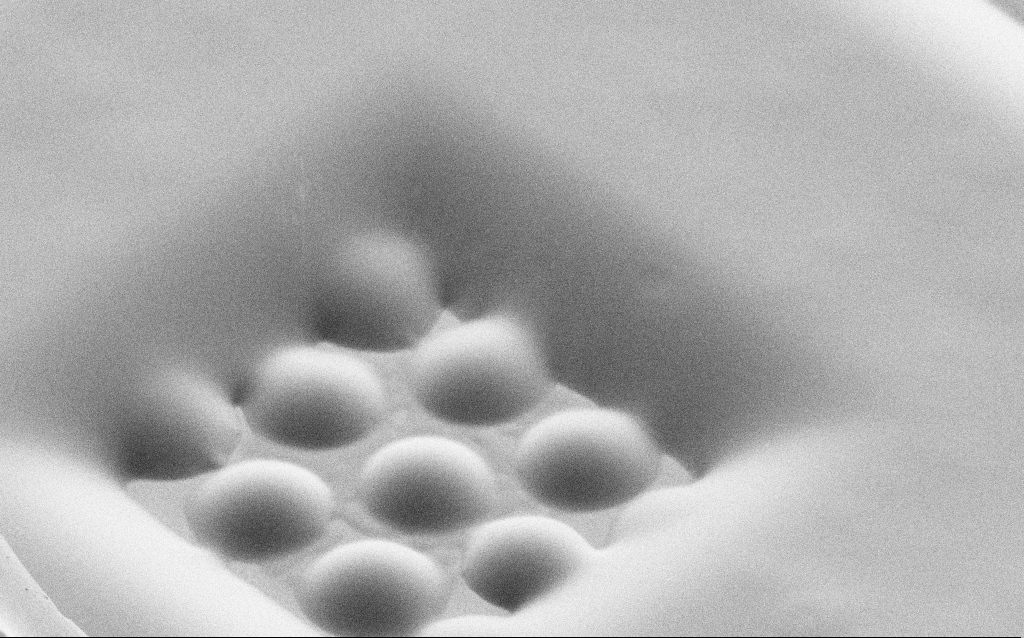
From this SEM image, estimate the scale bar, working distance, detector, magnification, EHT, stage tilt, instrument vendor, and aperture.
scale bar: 20000 nm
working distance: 6 mm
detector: SE2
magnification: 2.82 K X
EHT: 1.5 kV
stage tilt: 45°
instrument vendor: Zeiss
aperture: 30 µm